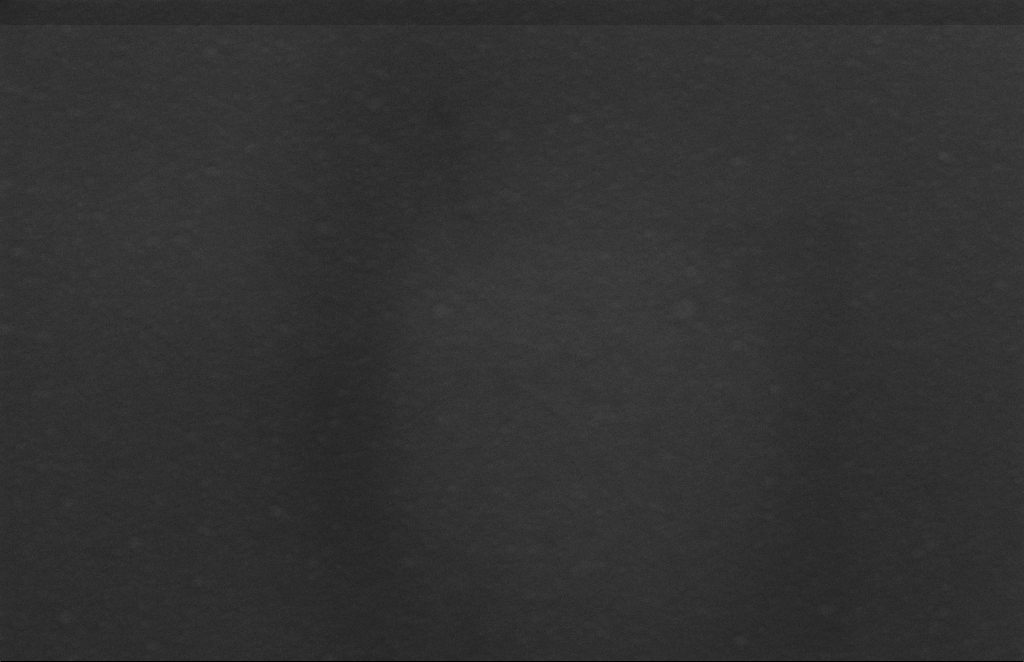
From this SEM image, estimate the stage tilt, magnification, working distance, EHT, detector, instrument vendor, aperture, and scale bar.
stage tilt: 0°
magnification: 205.38 K X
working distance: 5 mm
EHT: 5 kV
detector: InLens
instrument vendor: Zeiss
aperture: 20 µm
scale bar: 100 nm